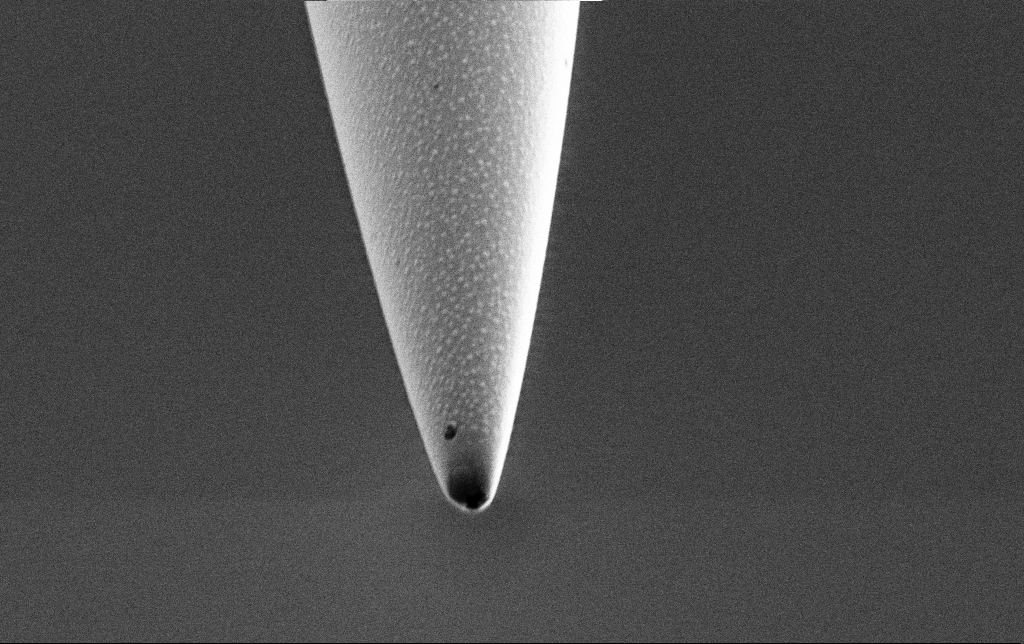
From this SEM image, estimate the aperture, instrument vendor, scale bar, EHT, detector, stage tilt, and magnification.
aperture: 30 µm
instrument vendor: Zeiss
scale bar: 2000 nm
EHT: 1 kV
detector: SE2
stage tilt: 45°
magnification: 15 K X